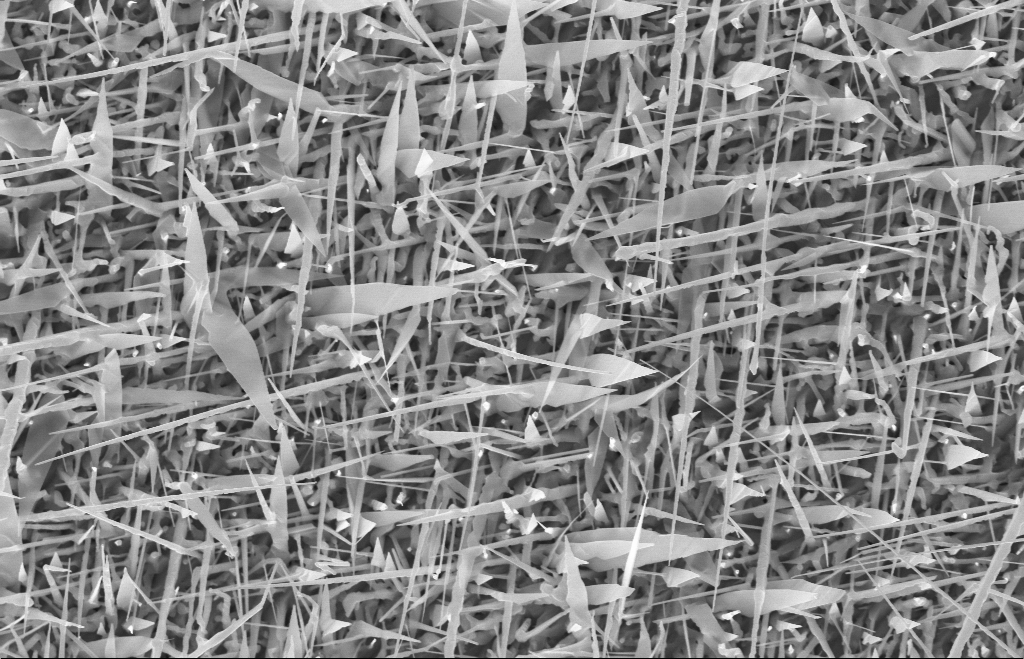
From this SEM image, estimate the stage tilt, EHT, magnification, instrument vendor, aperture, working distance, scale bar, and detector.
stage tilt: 0°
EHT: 10 kV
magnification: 20 K X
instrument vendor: Zeiss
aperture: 30 µm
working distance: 10 mm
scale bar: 1000 nm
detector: InLens